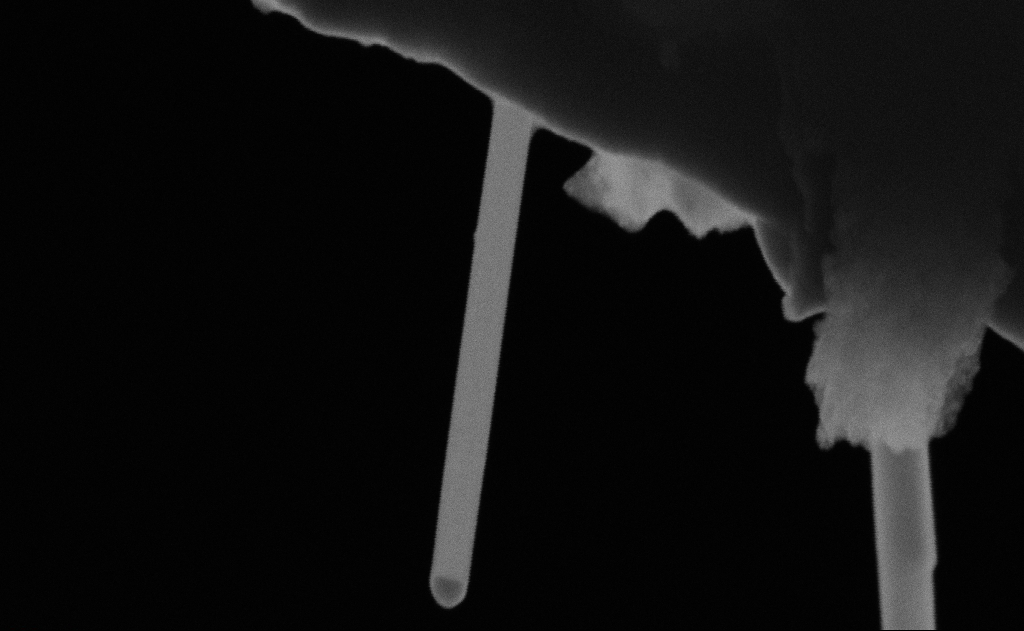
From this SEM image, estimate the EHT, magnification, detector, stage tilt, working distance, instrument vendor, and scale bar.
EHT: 20 kV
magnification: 256.16 K X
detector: SE2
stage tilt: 0°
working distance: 9 mm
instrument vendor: Zeiss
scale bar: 200 nm